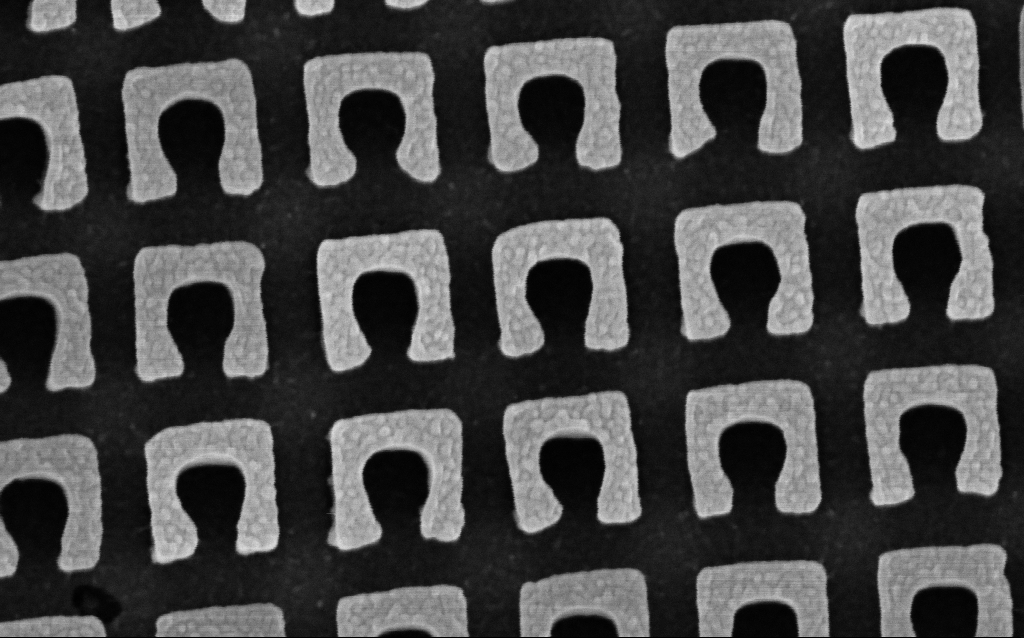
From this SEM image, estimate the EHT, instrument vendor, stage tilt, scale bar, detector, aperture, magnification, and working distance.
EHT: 3 kV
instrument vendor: Zeiss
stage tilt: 0°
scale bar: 200 nm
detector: InLens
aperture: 30 µm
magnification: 144.62 K X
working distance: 4.6 mm